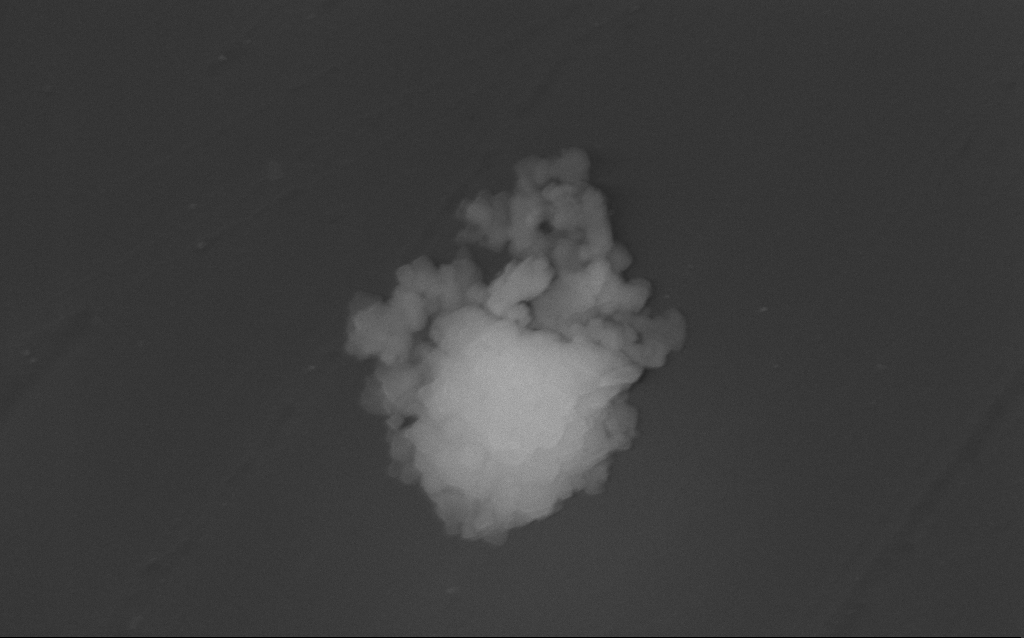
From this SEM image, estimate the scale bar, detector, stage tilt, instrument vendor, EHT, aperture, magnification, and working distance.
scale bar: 200 nm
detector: InLens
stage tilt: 20°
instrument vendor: Zeiss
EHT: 10 kV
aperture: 30 µm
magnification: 84.2 K X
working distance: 4 mm